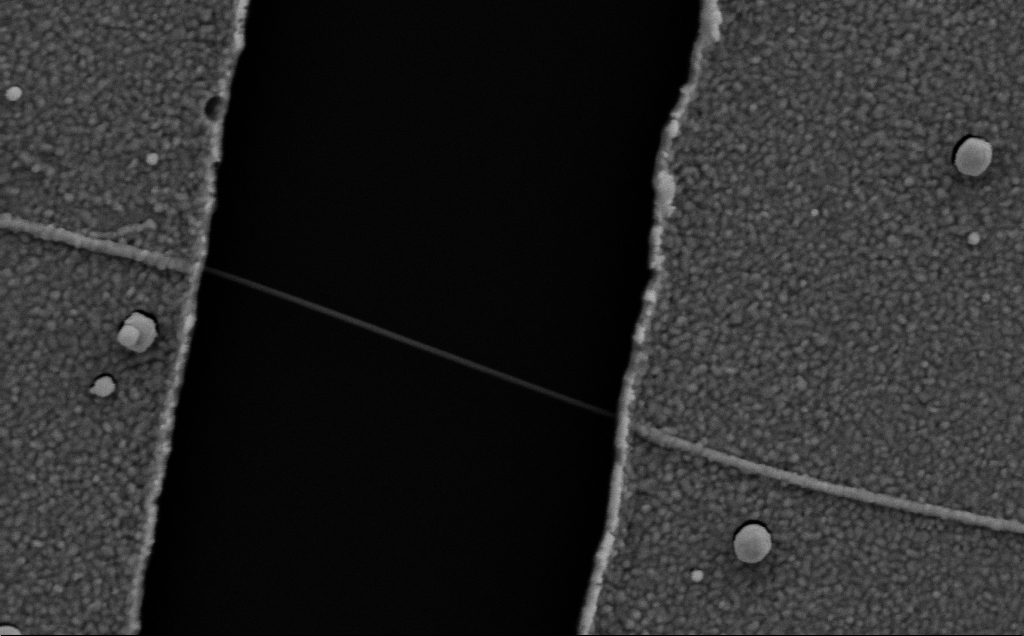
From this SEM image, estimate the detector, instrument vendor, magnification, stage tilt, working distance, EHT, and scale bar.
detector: SE2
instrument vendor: Zeiss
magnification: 90.76 K X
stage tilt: -0.7°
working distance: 8 mm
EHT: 5 kV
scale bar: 200 nm